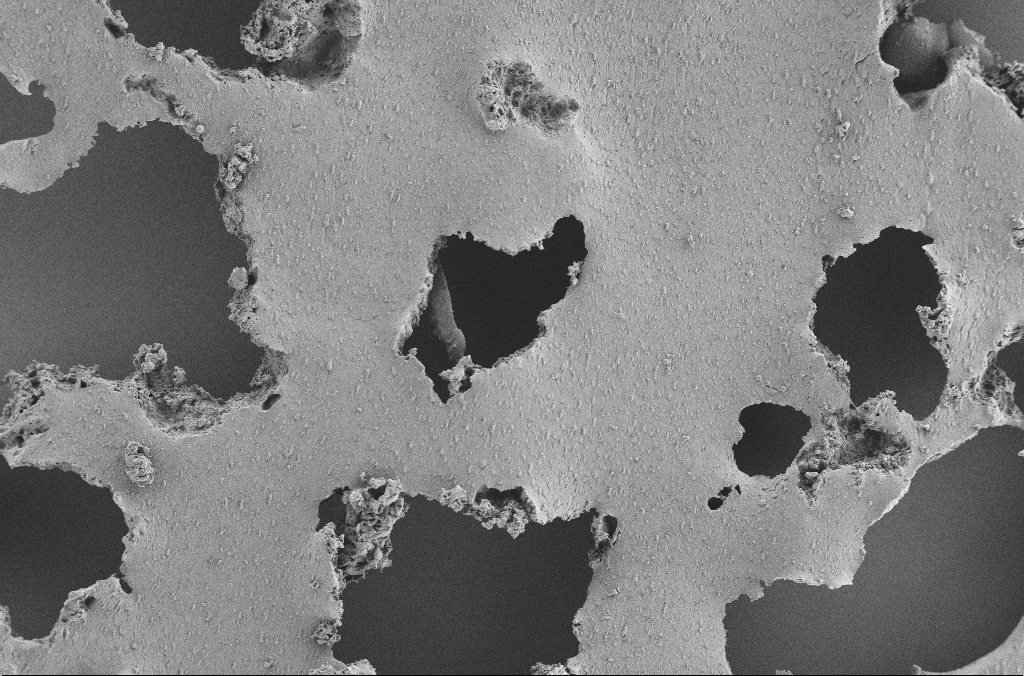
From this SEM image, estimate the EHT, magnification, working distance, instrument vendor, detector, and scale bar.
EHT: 2 kV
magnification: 0.25 K X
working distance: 3.6 mm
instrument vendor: Zeiss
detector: SE2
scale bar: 100000 nm